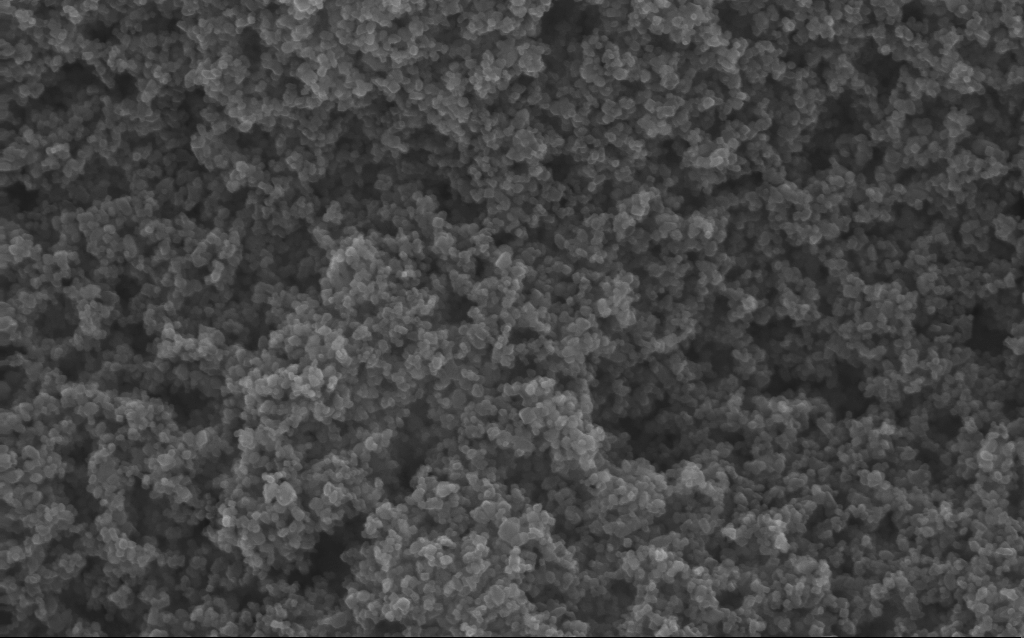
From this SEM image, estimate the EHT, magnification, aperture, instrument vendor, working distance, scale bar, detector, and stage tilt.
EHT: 10 kV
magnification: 130 K X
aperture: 30 µm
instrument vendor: Zeiss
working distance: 2.7 mm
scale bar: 100 nm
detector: InLens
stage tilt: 0°